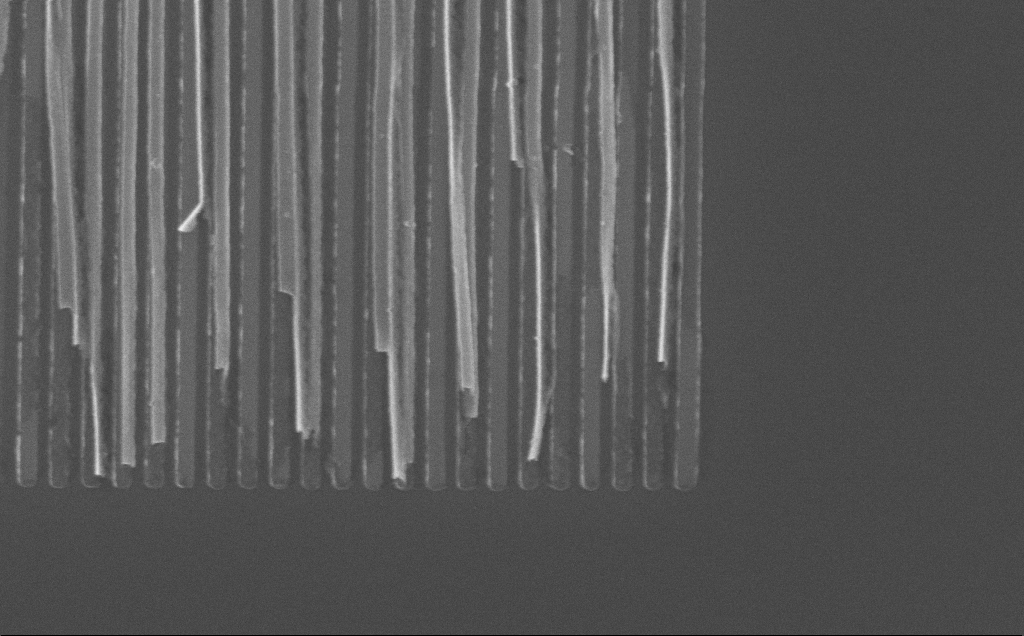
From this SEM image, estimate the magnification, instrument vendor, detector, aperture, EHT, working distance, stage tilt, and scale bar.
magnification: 57.52 K X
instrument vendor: Zeiss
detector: InLens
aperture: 30 µm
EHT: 10 kV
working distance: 7 mm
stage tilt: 0°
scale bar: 1000 nm